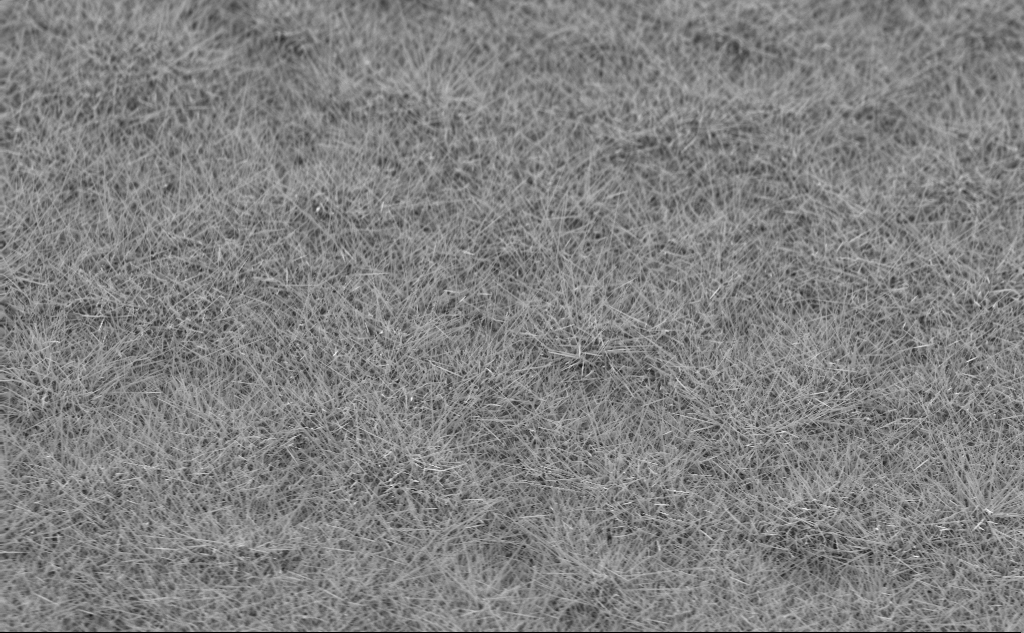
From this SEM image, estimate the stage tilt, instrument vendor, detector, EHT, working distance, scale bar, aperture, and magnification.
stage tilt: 45°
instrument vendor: Zeiss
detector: InLens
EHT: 10 kV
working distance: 4 mm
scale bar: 10000 nm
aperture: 30 µm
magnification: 5 K X